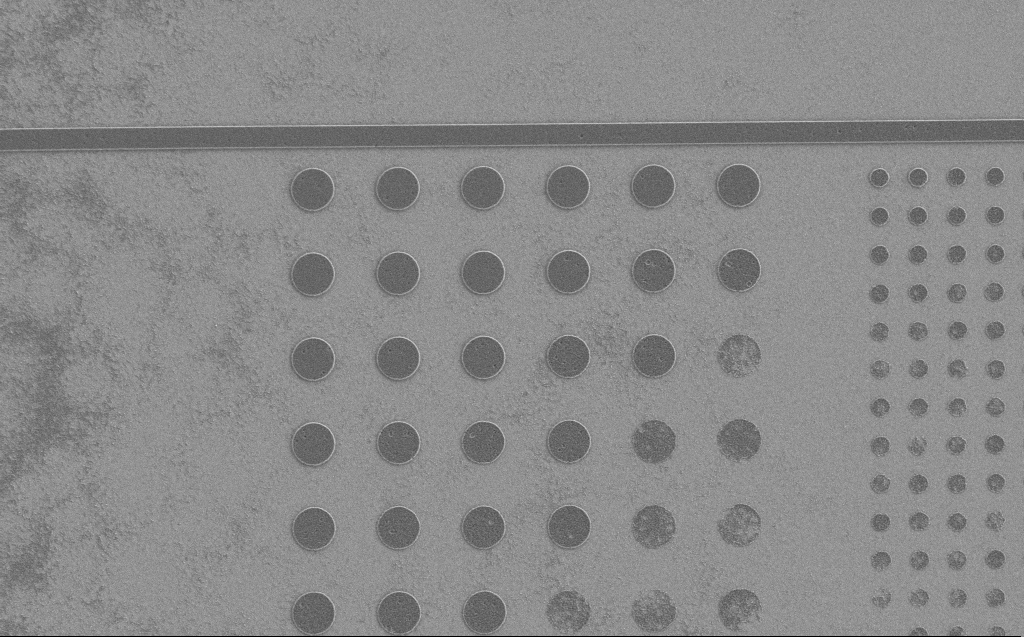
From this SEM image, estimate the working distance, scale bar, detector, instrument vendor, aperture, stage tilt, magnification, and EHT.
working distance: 5 mm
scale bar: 2000 nm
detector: SE2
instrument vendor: Zeiss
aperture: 30 µm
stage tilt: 0°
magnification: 7.86 K X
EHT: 1.2 kV